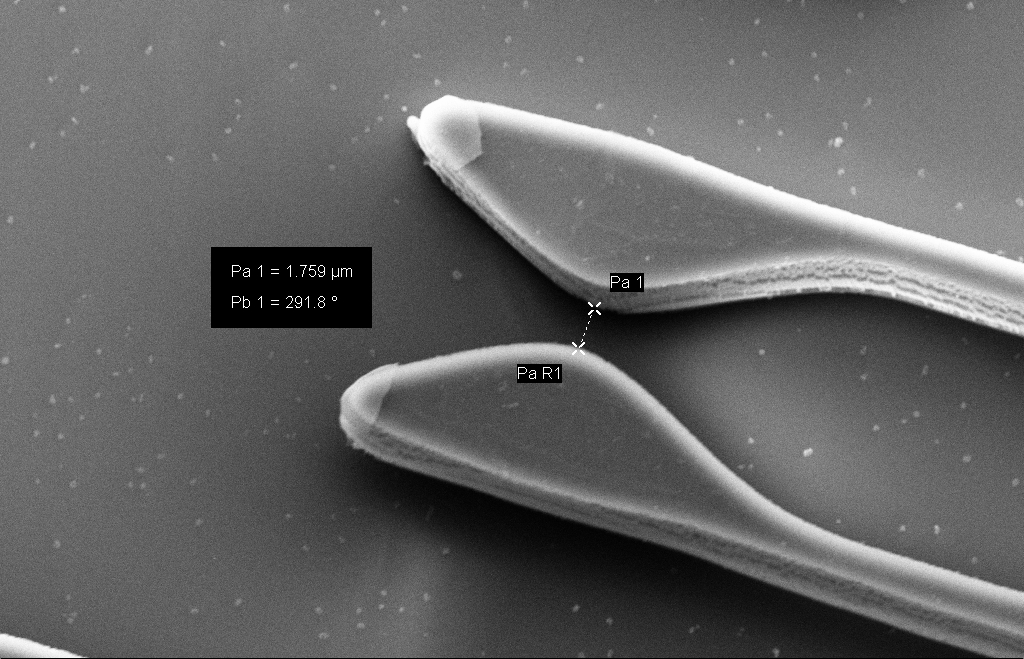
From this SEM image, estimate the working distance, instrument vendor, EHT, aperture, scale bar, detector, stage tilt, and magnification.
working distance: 11 mm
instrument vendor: Zeiss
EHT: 10 kV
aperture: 30 µm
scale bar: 2000 nm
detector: SE2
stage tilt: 15.3°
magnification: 9 K X